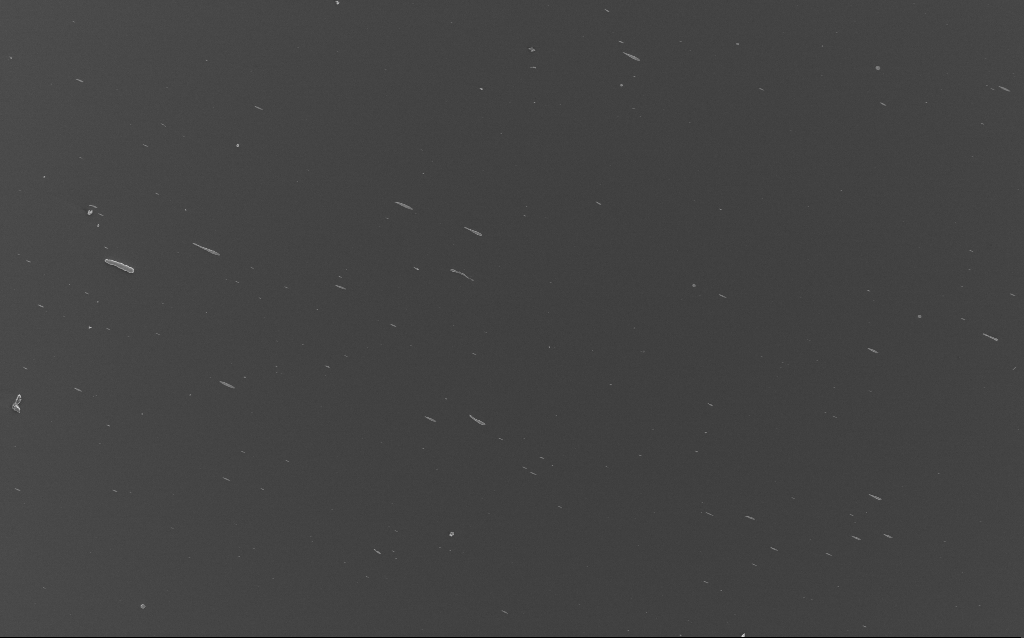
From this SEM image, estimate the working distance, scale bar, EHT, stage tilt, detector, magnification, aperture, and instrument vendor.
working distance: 3 mm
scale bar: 20000 nm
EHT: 3 kV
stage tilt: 0°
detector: InLens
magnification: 1.1 K X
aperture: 30 µm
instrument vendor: Zeiss